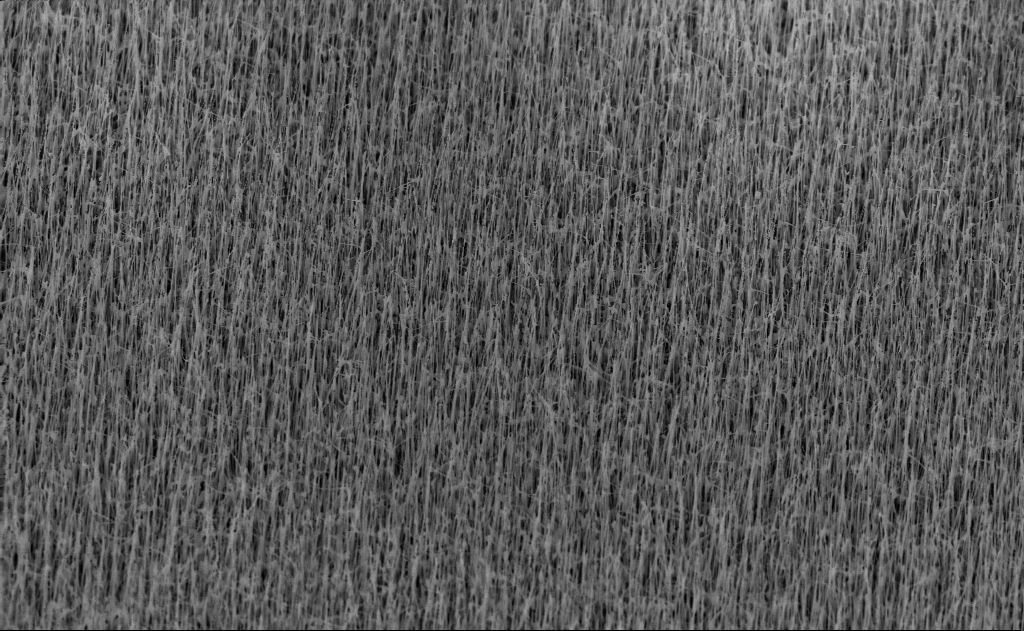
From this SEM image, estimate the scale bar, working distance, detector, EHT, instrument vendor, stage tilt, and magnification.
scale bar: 2000 nm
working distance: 7 mm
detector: InLens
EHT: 10 kV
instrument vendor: Zeiss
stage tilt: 45°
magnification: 10 K X